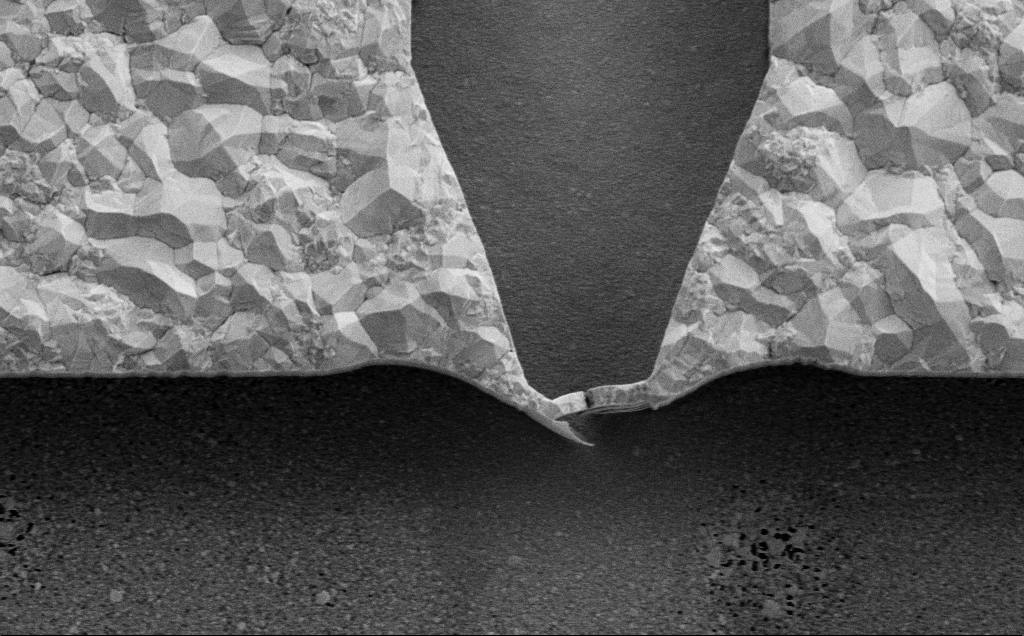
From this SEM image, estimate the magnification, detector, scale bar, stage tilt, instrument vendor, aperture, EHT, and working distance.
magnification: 13.11 K X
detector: SE2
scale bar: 2000 nm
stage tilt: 0°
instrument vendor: Zeiss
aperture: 30 µm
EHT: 5 kV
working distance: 11 mm